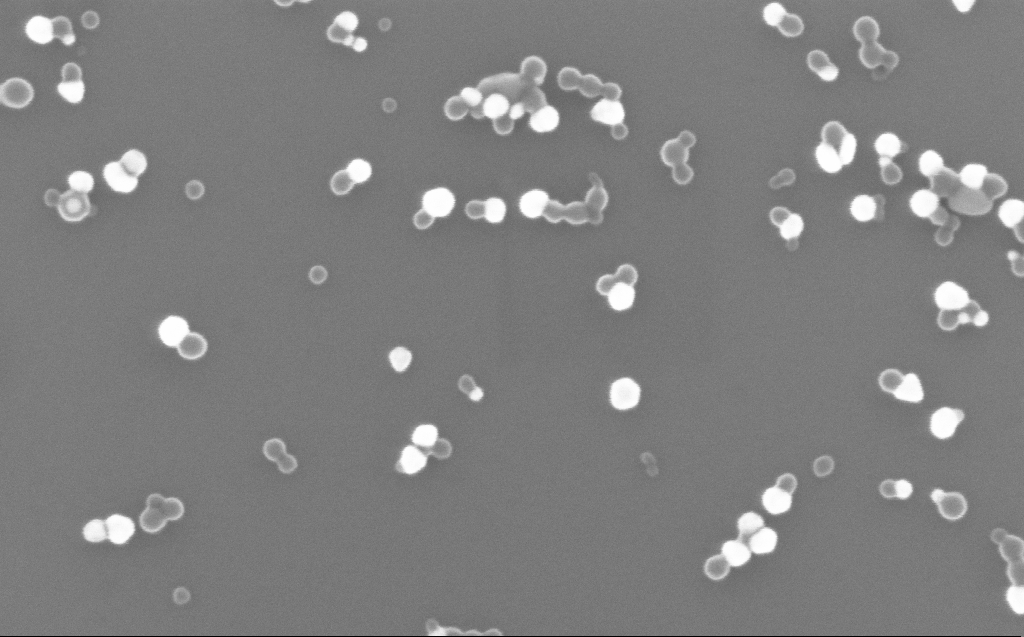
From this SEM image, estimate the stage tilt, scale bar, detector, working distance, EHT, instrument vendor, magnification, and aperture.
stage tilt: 0°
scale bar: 200 nm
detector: InLens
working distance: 3 mm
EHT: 10 kV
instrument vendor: Zeiss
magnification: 200 K X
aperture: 30 µm